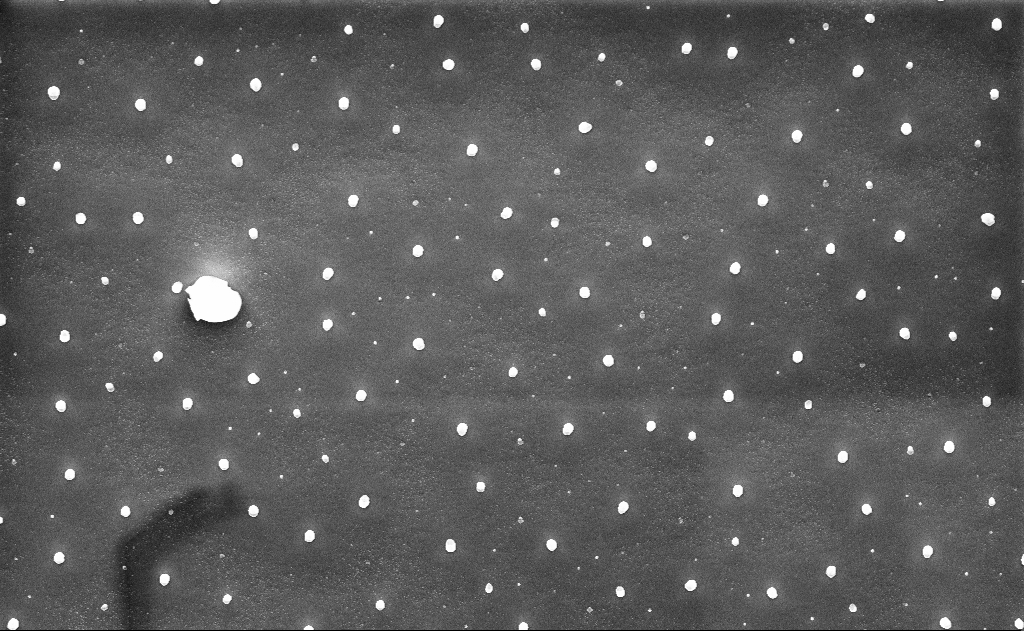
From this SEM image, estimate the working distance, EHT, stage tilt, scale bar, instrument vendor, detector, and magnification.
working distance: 10 mm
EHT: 10 kV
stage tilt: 0°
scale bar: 2000 nm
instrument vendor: Zeiss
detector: InLens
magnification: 10 K X